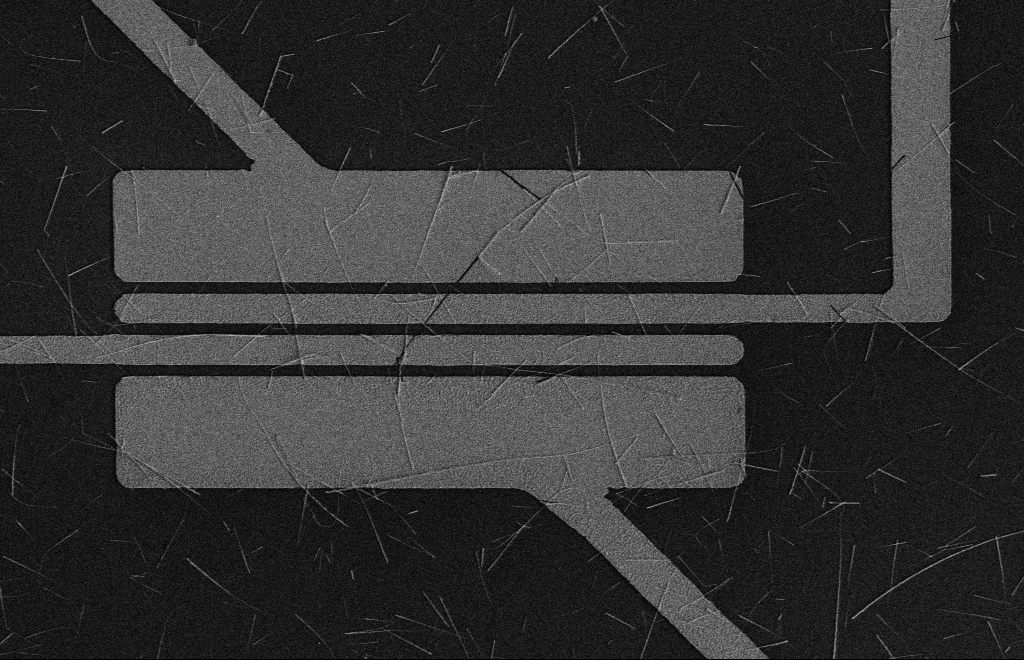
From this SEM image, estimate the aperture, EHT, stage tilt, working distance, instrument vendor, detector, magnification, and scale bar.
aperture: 10 µm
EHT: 5 kV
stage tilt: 0°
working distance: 16 mm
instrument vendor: Zeiss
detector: SE2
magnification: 3.81 K X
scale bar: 10000 nm